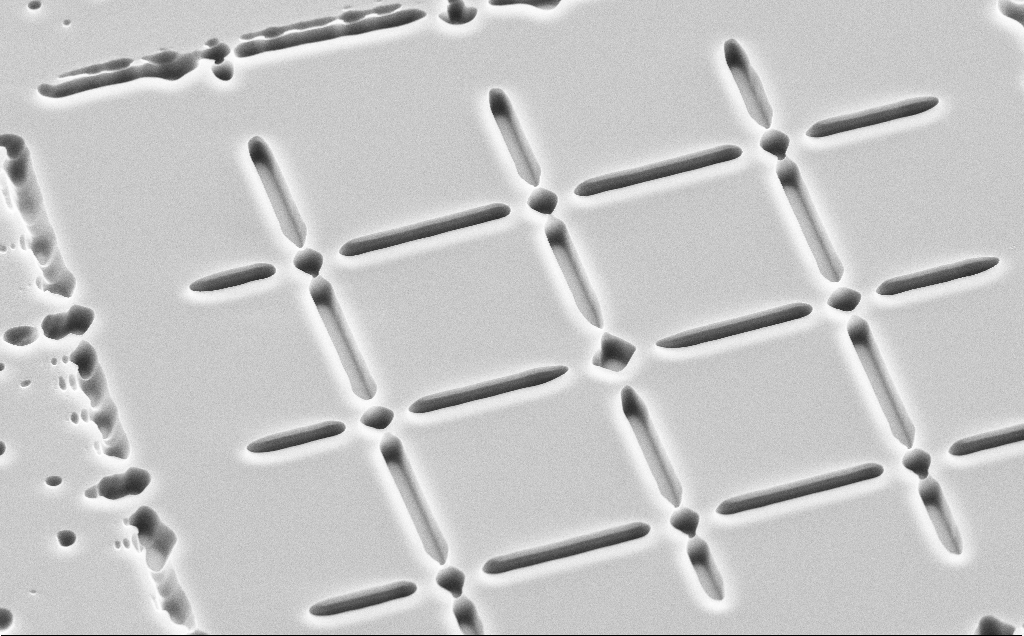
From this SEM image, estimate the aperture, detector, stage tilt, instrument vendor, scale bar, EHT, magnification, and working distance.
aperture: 30 µm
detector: SE2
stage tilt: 45°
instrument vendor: Zeiss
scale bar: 10000 nm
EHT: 10 kV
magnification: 4.42 K X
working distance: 11 mm